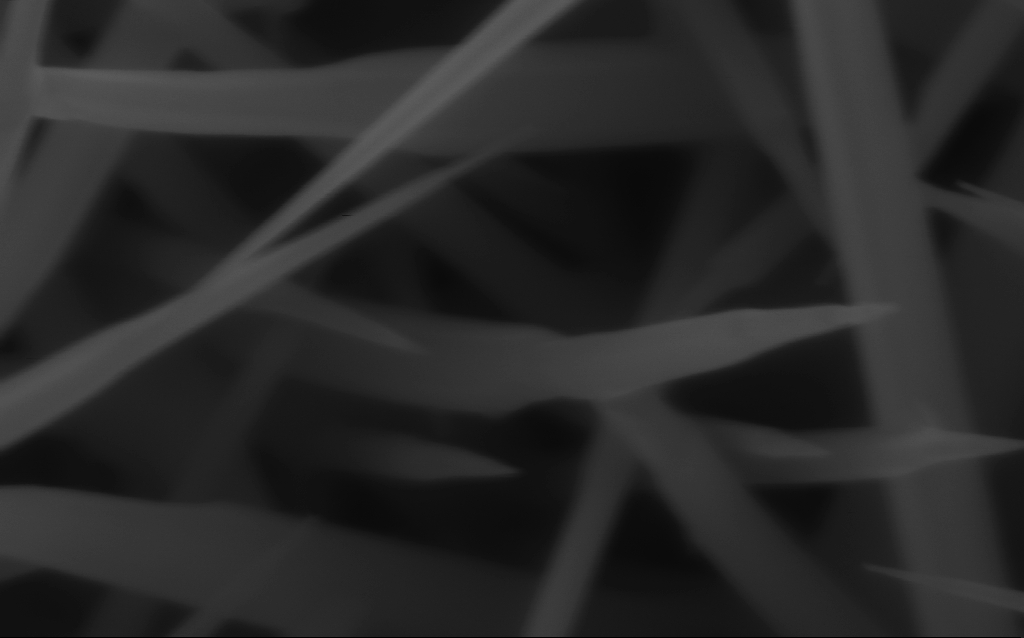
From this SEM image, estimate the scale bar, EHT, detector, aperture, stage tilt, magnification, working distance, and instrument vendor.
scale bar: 200 nm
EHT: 10 kV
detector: InLens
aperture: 30 µm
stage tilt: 0°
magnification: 191.6 K X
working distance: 6 mm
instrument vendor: Zeiss